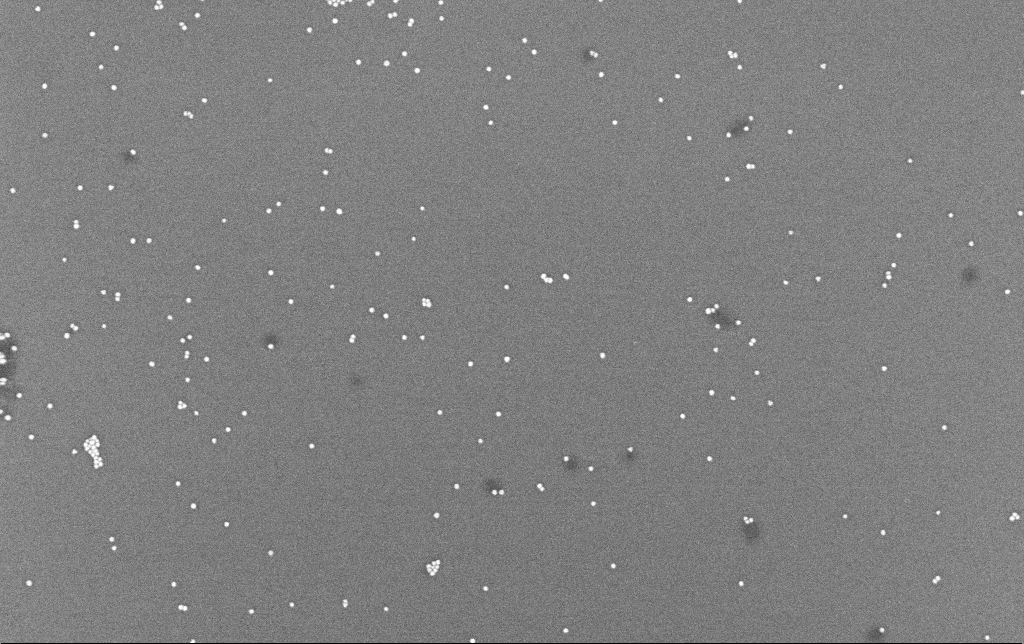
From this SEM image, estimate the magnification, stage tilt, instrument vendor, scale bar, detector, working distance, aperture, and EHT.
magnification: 100 K X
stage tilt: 0°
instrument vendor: Zeiss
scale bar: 200 nm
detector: InLens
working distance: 6.6 mm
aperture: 30 µm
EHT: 8 kV